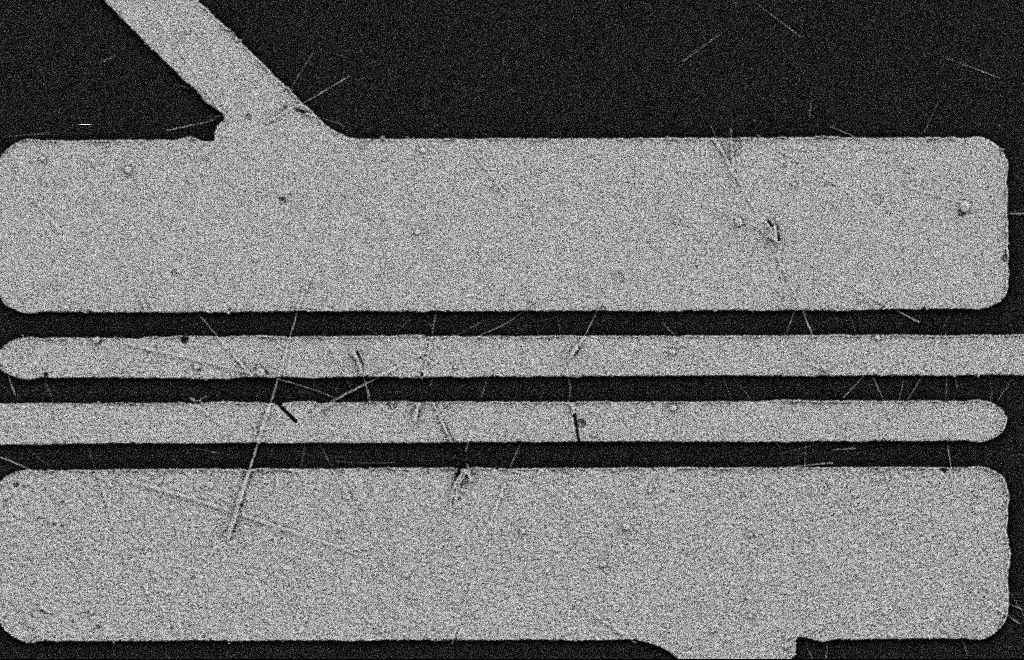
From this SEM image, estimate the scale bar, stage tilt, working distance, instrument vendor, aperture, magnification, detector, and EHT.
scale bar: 2000 nm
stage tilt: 0°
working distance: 11 mm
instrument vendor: Zeiss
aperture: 20 µm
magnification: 6.02 K X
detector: SE2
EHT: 2 kV